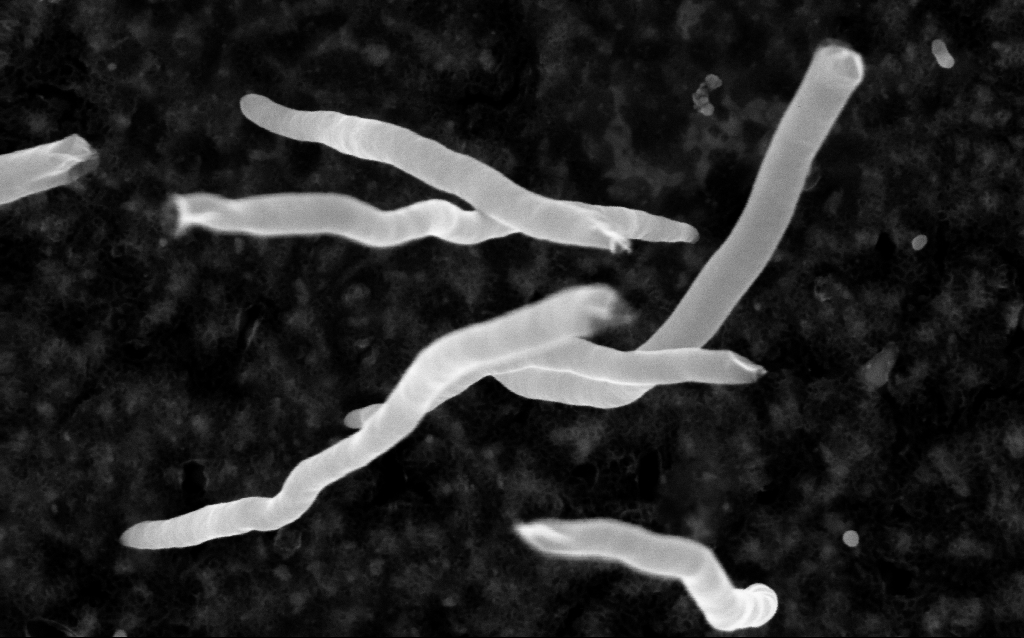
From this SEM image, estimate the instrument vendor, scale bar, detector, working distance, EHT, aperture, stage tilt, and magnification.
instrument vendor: Zeiss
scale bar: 100 nm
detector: InLens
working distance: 2 mm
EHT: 5 kV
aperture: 30 µm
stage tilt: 0°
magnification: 200 K X